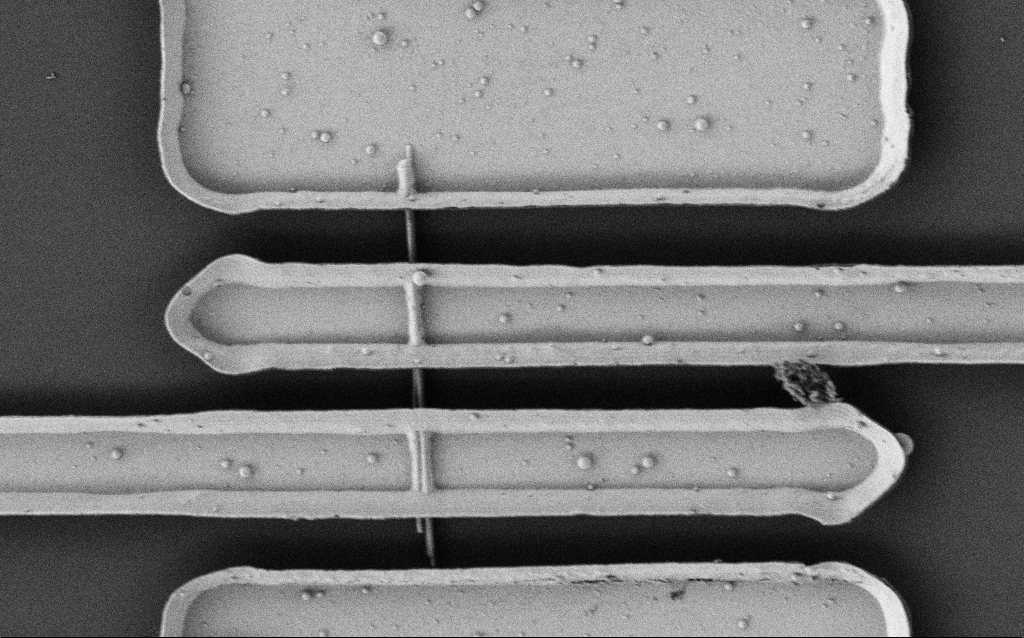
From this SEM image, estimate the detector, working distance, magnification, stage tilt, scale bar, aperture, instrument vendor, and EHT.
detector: SE2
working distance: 7.7 mm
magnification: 8.9 K X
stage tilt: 0°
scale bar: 2000 nm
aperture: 30 µm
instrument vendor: Zeiss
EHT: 2 kV